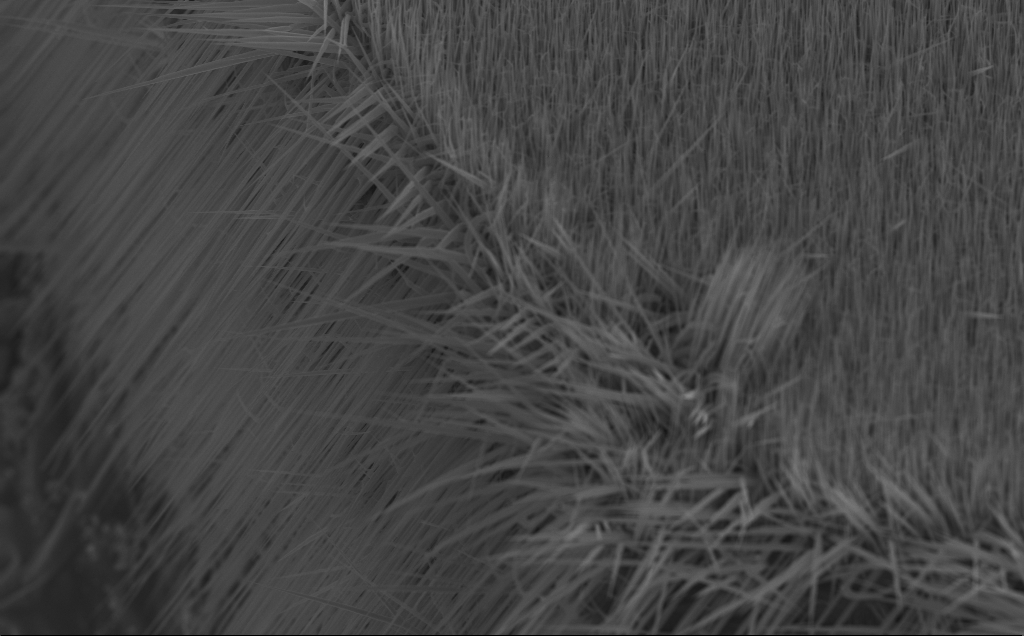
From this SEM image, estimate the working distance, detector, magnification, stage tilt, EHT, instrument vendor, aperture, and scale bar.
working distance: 7 mm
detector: InLens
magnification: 18.91 K X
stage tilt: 43.6°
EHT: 10 kV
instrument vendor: Zeiss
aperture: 30 µm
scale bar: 2000 nm